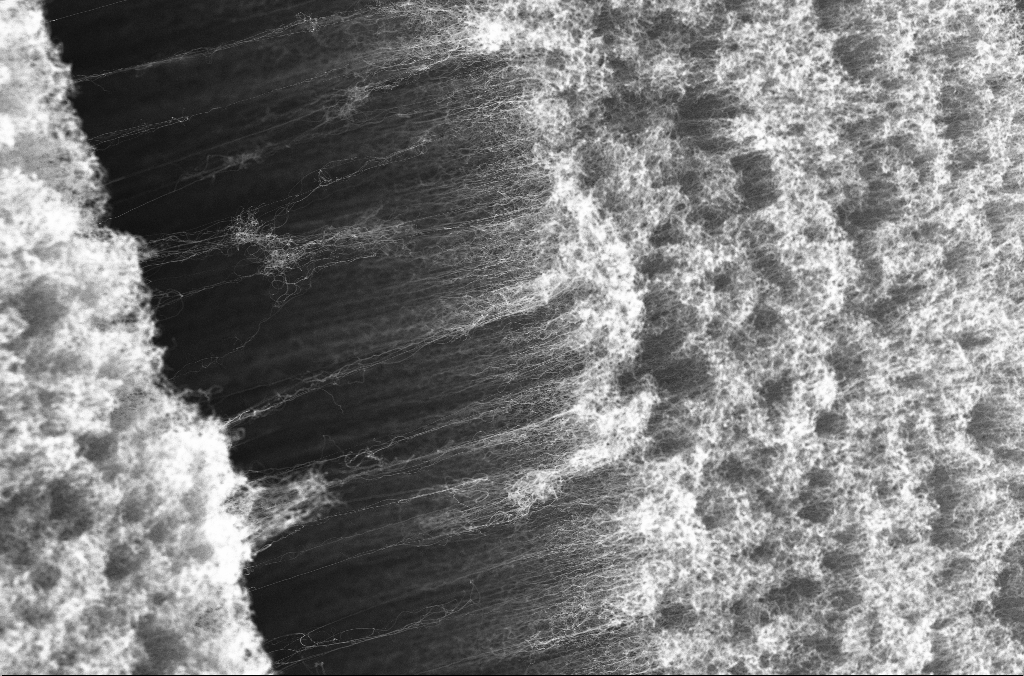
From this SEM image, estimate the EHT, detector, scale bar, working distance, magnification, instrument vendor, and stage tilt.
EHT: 5 kV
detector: InLens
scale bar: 2000 nm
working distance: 3.9 mm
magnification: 10 K X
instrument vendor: Zeiss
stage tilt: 0°